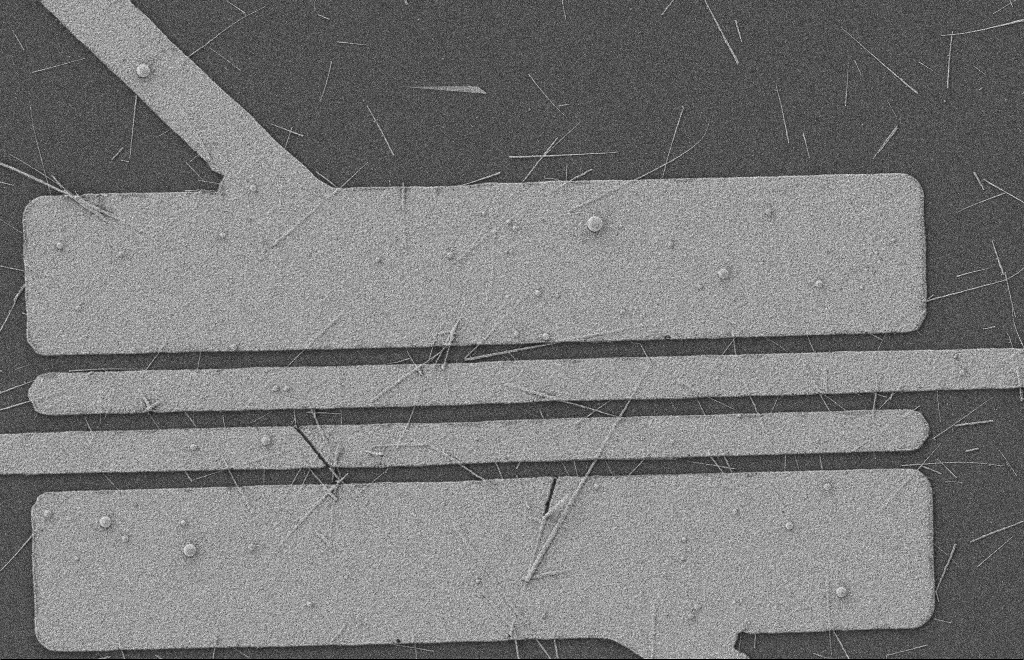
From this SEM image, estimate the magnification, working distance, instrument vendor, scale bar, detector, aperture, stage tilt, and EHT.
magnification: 5.4 K X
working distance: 8 mm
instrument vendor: Zeiss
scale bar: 2000 nm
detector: SE2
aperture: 20 µm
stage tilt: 0°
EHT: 2 kV